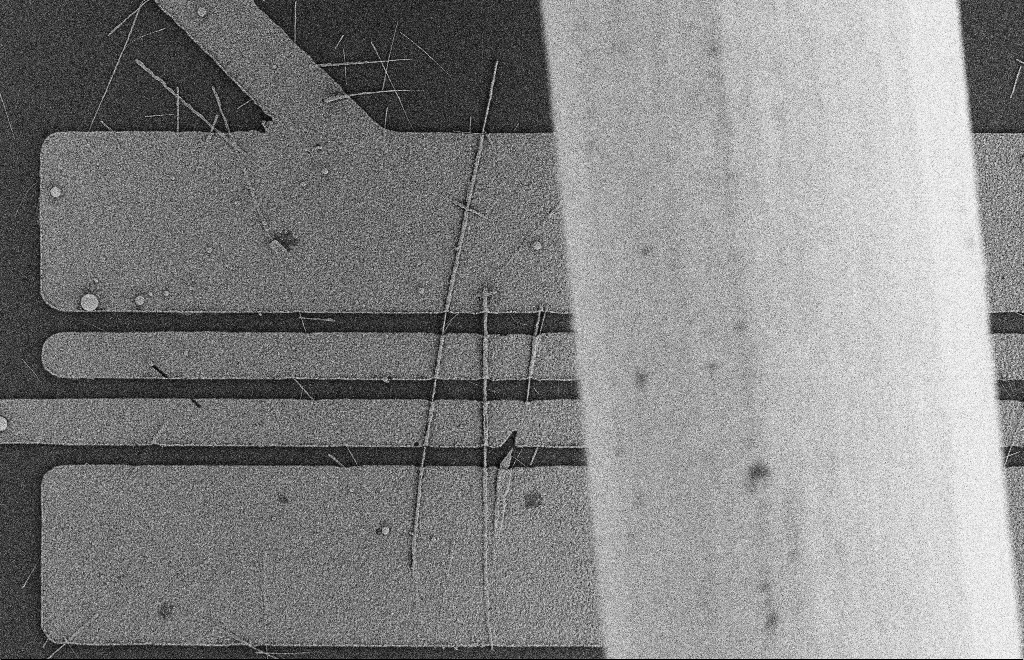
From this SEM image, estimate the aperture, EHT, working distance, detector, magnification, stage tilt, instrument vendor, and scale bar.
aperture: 20 µm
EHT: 2 kV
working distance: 9 mm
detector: SE2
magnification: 6.15 K X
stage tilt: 0°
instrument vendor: Zeiss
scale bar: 2000 nm